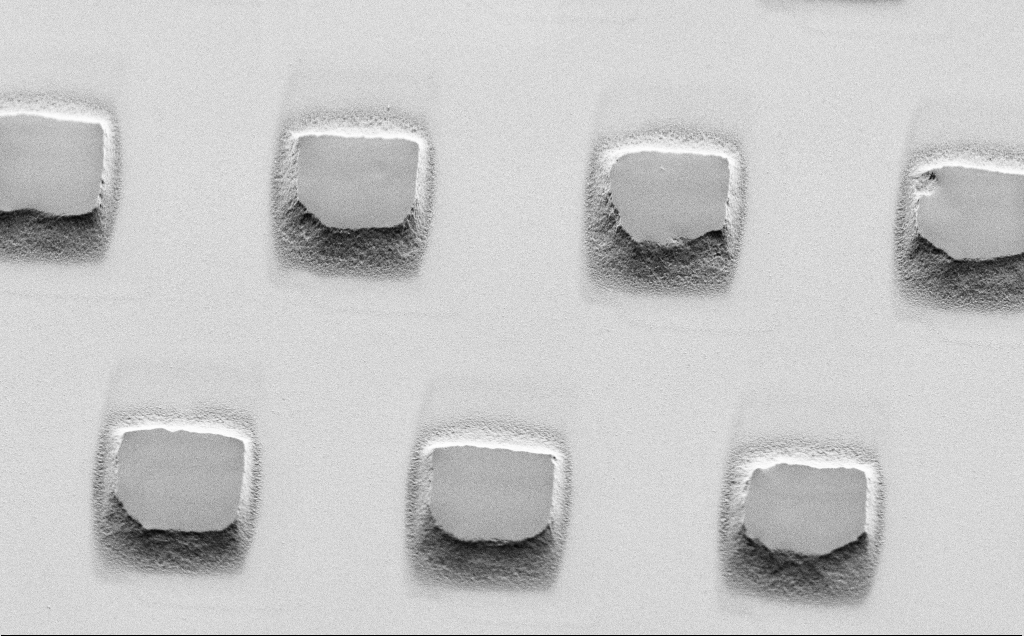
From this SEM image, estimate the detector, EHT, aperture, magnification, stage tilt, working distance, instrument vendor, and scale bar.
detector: SE2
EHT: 1.5 kV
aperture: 30 µm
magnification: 5.74 K X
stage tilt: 45°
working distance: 7 mm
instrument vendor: Zeiss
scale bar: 10000 nm